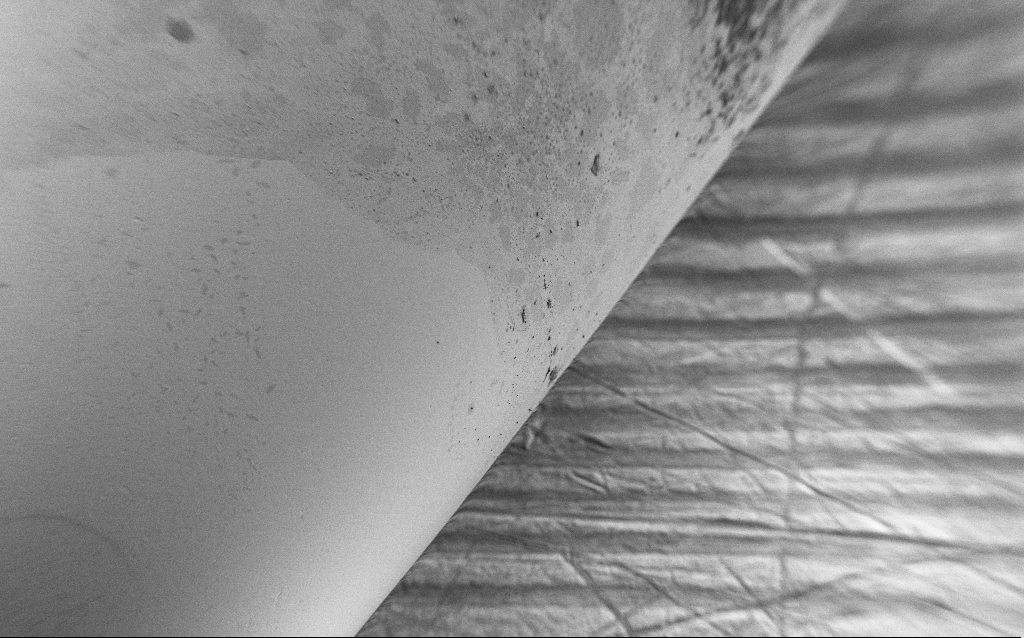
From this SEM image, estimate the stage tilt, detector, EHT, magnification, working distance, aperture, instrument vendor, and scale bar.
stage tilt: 45°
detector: SE2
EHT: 1 kV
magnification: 0.25 K X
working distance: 7.7 mm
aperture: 30 µm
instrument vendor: Zeiss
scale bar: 100000 nm